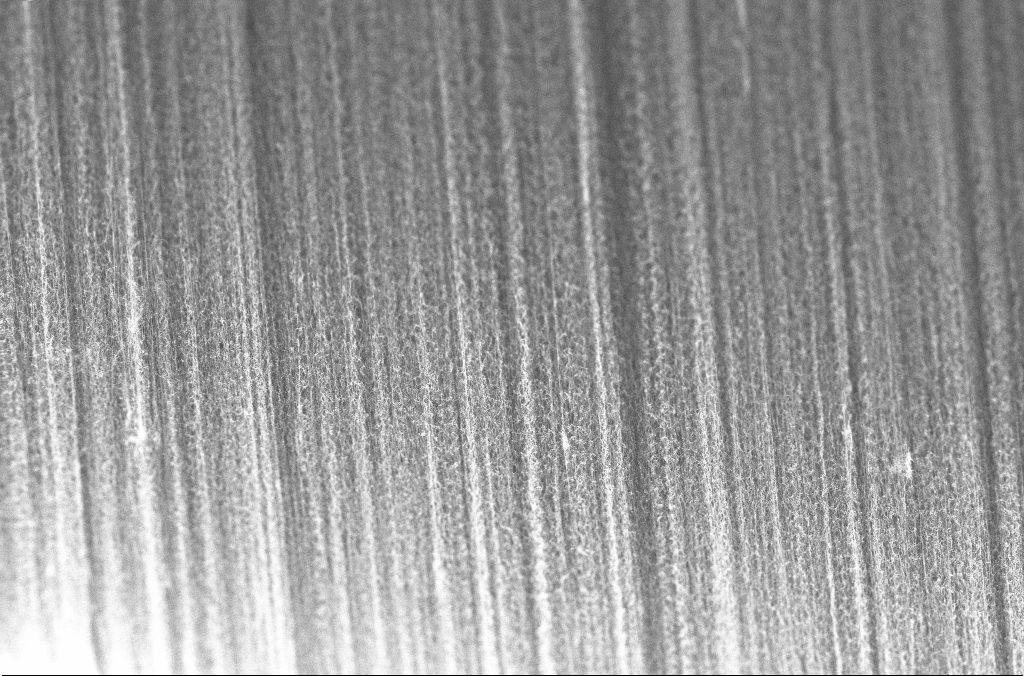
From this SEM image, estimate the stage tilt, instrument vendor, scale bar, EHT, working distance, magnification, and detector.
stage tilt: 0°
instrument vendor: Zeiss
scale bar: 10000 nm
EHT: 20 kV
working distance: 4 mm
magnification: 5 K X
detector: InLens